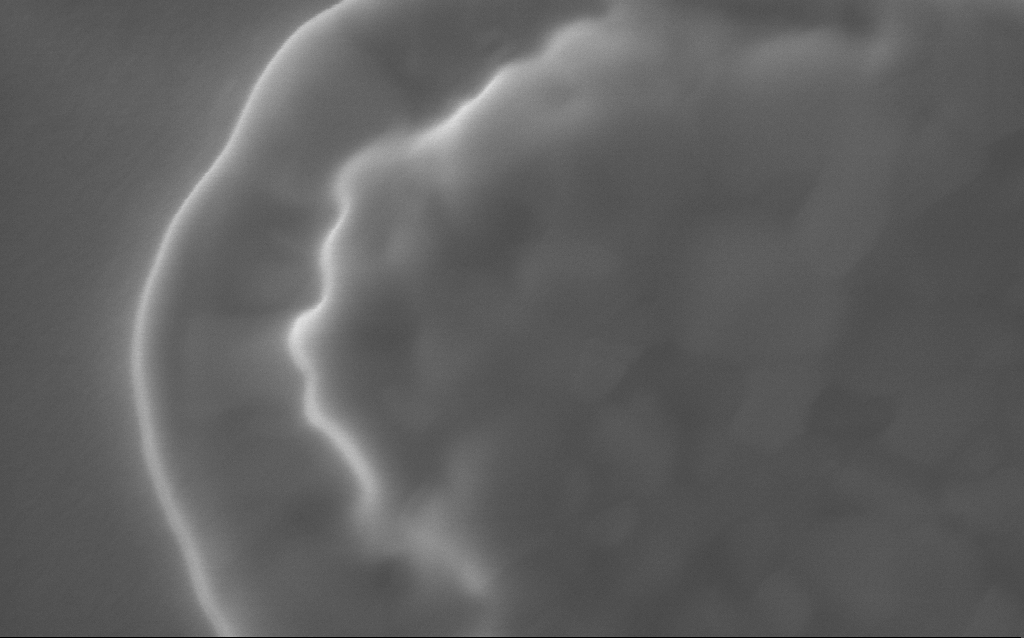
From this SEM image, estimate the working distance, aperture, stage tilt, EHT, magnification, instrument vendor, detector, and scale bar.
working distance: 2 mm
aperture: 30 µm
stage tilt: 0°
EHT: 5 kV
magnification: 232.44 K X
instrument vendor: Zeiss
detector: InLens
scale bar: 200 nm